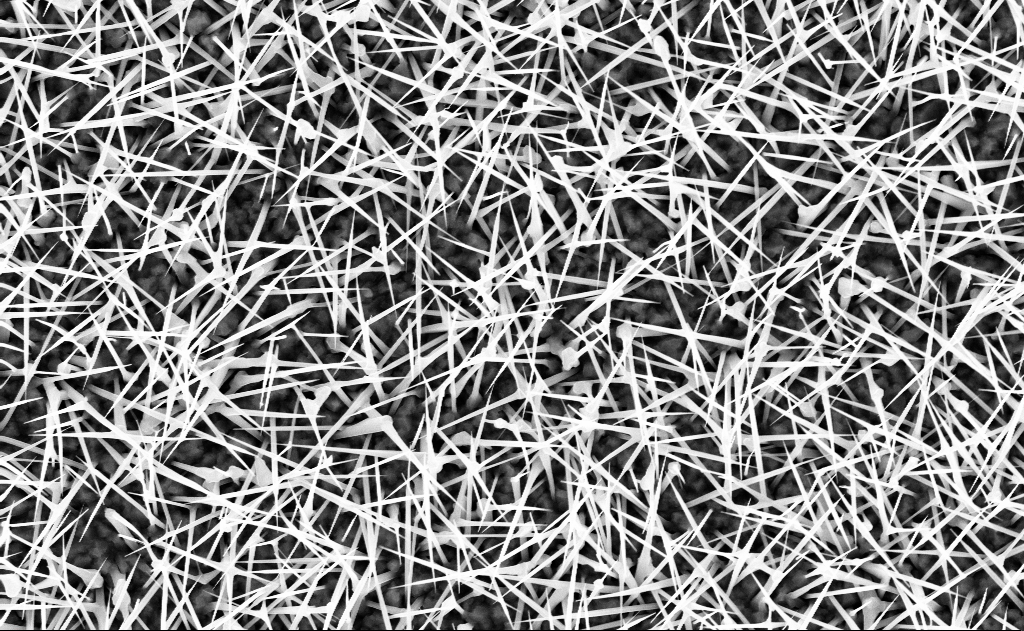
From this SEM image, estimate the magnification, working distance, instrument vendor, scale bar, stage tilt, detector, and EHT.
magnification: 20 K X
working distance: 9 mm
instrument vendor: Zeiss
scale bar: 2000 nm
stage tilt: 0°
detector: InLens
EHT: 10 kV